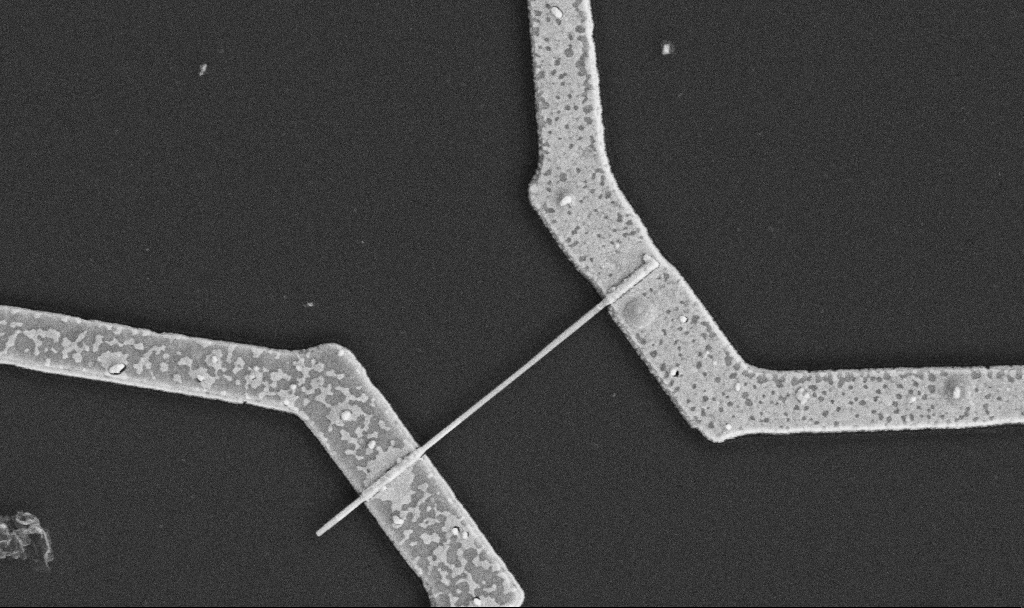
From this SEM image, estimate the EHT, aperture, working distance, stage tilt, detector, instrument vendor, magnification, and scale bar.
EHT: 5 kV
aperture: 30 µm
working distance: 10.6 mm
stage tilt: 0°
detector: SE2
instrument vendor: Zeiss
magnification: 30 K X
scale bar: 1000 nm